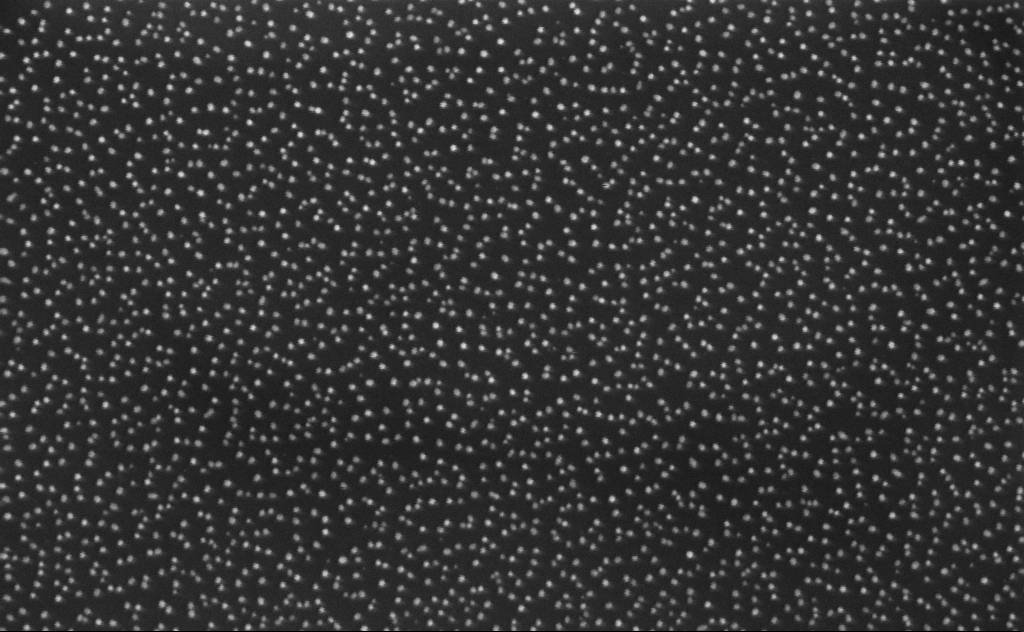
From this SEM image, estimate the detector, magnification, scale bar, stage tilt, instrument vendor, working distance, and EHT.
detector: InLens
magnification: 150 K X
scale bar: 100 nm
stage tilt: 0°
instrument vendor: Zeiss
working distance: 7 mm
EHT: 3 kV